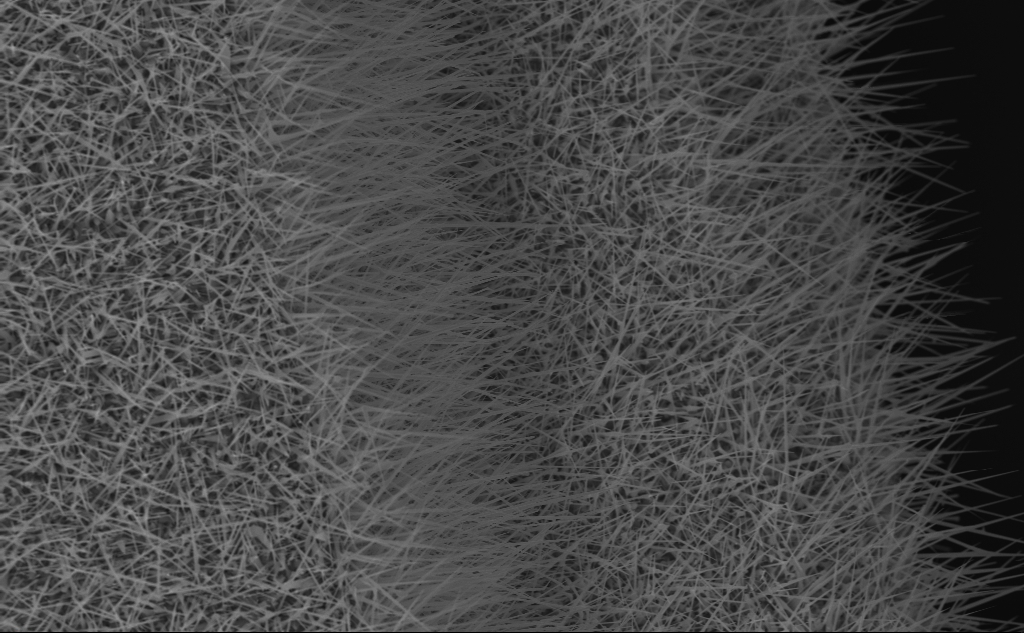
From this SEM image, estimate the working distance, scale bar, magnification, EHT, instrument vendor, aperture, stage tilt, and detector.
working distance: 7 mm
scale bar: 2000 nm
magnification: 10.14 K X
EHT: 10 kV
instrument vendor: Zeiss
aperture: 30 µm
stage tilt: -0°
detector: InLens